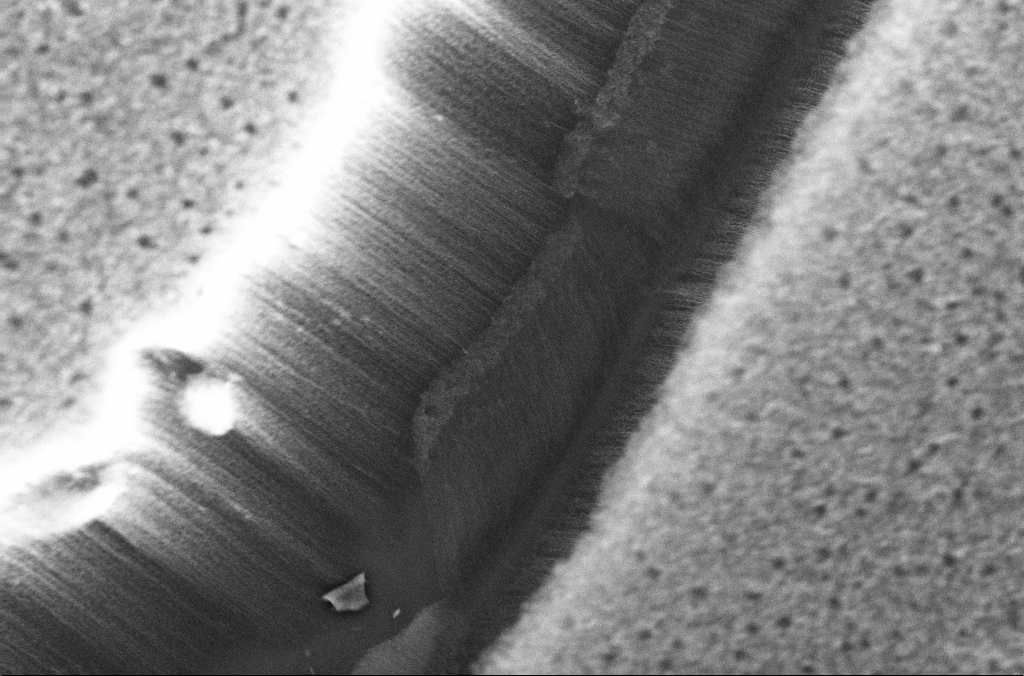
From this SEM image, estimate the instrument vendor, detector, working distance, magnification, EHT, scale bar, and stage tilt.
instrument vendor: Zeiss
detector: InLens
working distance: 4 mm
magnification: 5 K X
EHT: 10 kV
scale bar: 10000 nm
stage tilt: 0°